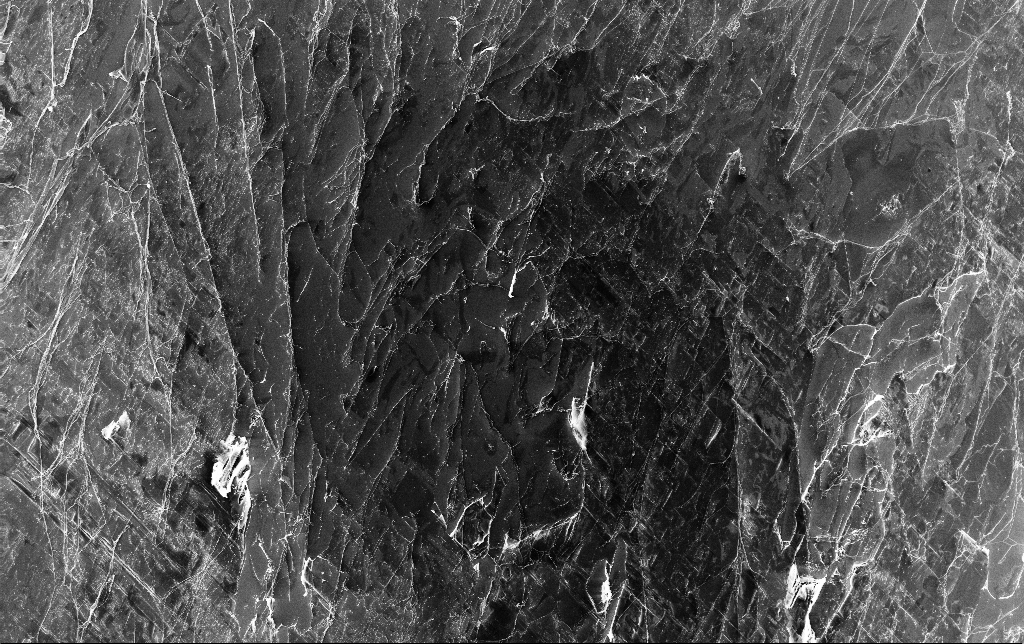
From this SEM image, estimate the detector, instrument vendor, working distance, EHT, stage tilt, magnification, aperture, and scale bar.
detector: InLens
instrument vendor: Zeiss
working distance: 3.4 mm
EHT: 10 kV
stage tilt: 0°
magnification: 0.483 K X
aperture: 30 µm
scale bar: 100000 nm